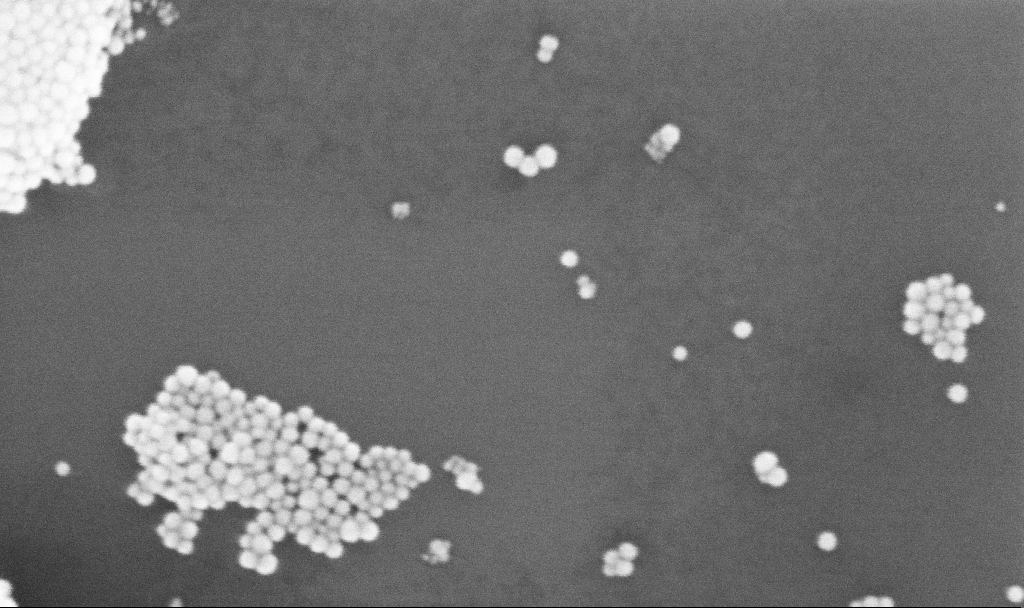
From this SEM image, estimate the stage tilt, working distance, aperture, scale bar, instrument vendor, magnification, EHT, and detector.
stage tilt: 0°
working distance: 3.1 mm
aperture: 30 µm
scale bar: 100 nm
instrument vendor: Zeiss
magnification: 354.18 K X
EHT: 10 kV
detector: InLens